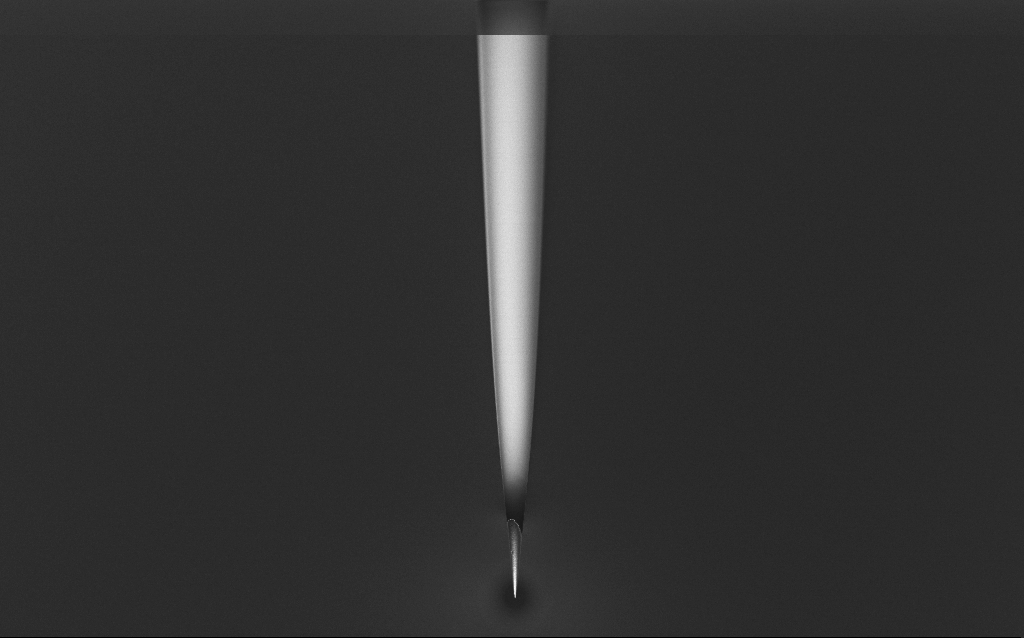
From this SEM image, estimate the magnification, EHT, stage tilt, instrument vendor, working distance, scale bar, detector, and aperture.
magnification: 1 K X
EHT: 1 kV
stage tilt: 45°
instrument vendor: Zeiss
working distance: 6 mm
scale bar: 20000 nm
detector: InLens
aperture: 30 µm